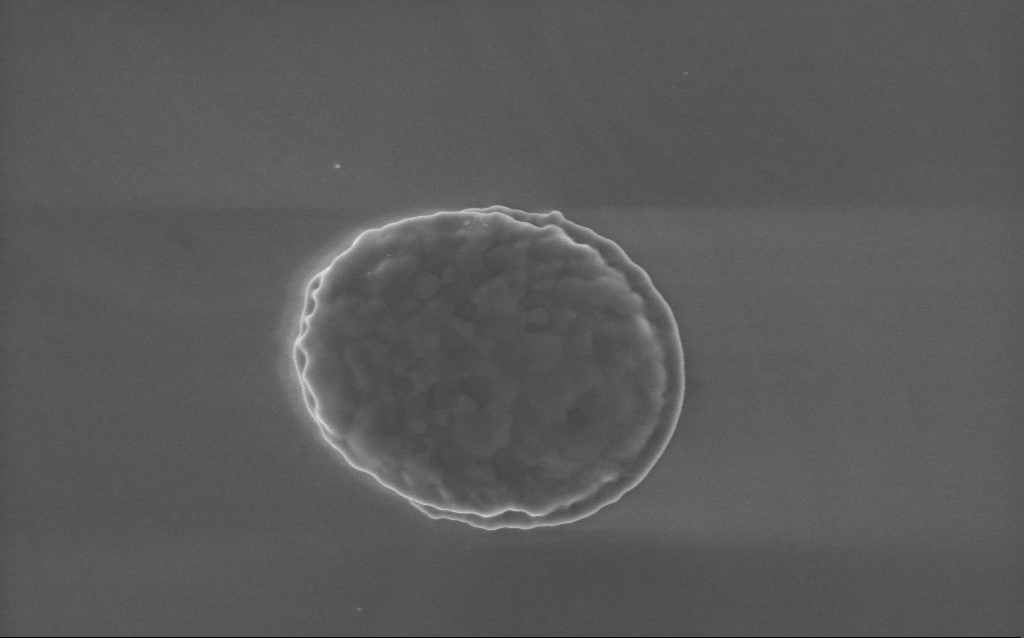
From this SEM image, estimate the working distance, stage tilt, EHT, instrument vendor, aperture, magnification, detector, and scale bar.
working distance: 4 mm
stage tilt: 0°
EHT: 5 kV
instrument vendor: Zeiss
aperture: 30 µm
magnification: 44 K X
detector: InLens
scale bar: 1000 nm